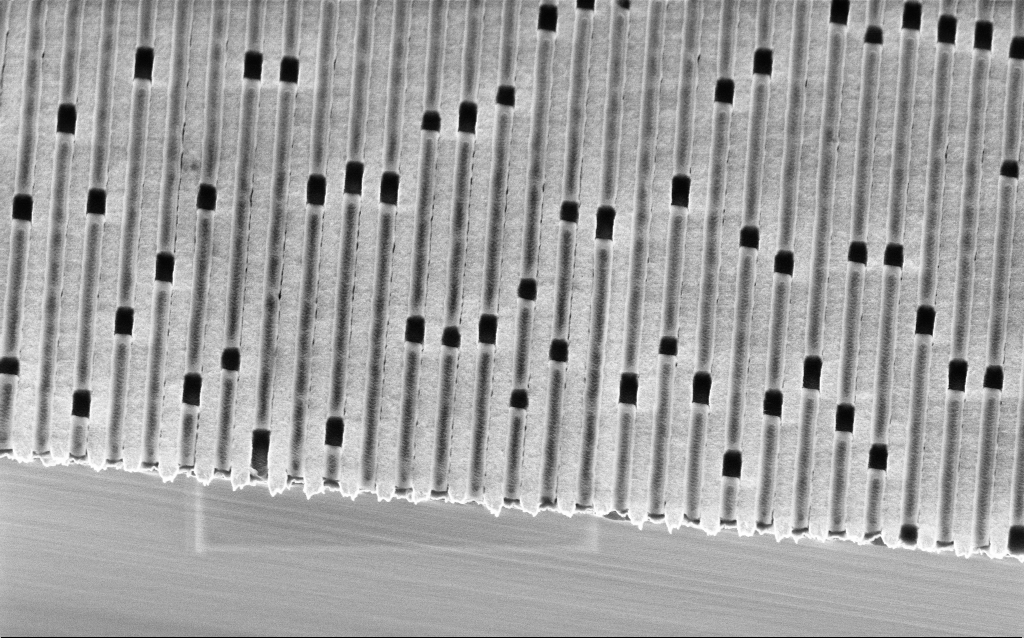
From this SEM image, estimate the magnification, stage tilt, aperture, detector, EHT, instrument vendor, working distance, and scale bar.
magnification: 26.8 K X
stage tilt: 32°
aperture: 30 µm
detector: InLens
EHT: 2 kV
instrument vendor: Zeiss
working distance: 4.1 mm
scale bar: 2000 nm